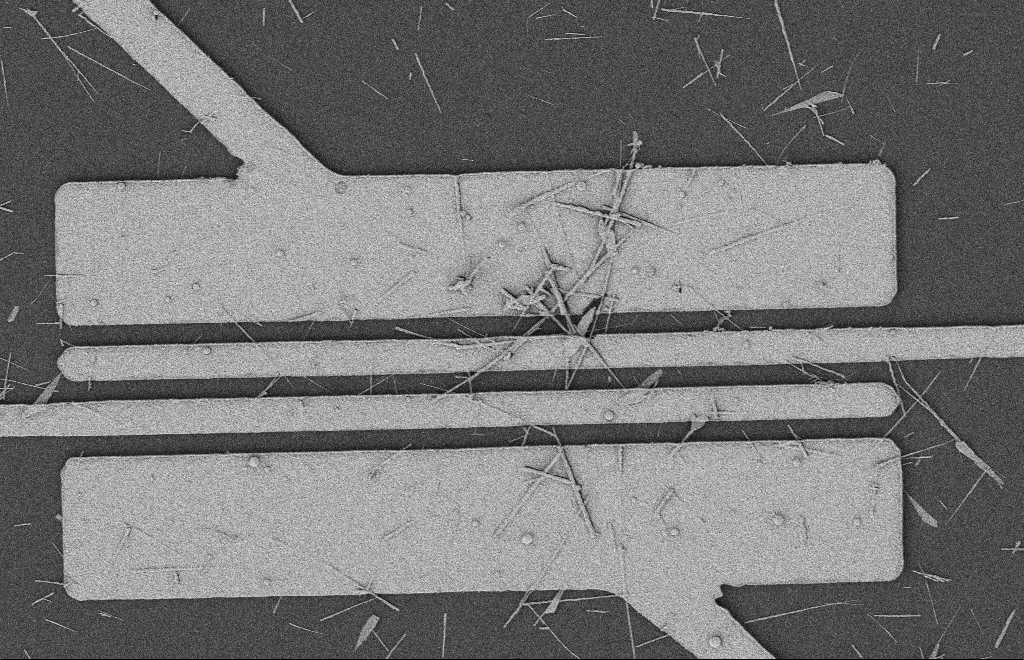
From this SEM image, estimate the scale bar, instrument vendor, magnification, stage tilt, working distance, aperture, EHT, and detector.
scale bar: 2000 nm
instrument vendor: Zeiss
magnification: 5.01 K X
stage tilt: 0°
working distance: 12 mm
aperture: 20 µm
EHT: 2 kV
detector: SE2